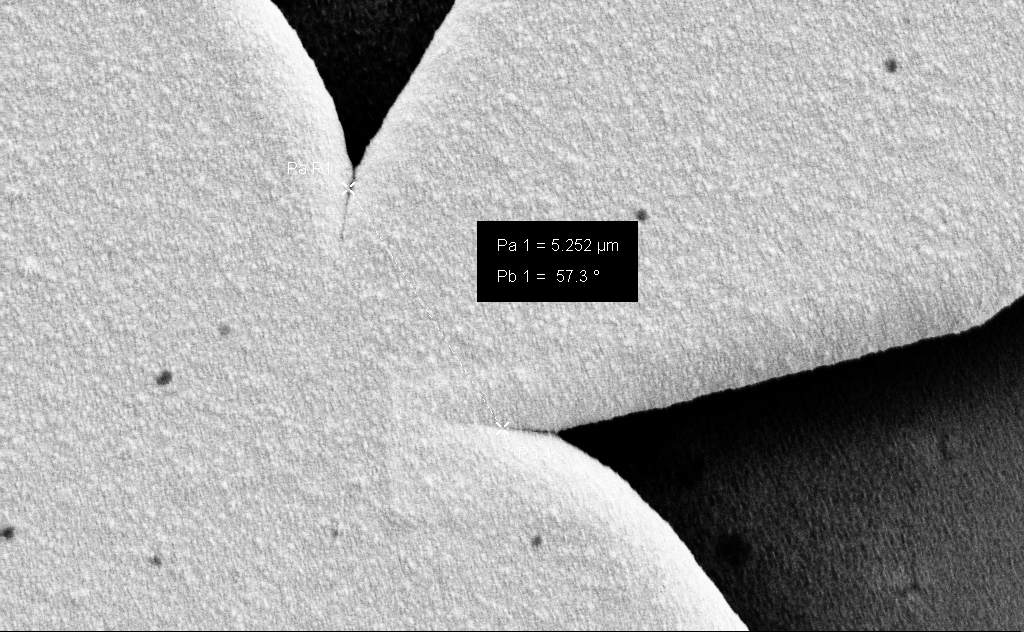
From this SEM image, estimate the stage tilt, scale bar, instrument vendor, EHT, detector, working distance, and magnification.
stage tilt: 0°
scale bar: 2000 nm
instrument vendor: Zeiss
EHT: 3 kV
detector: SE2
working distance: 13 mm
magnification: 19.94 K X